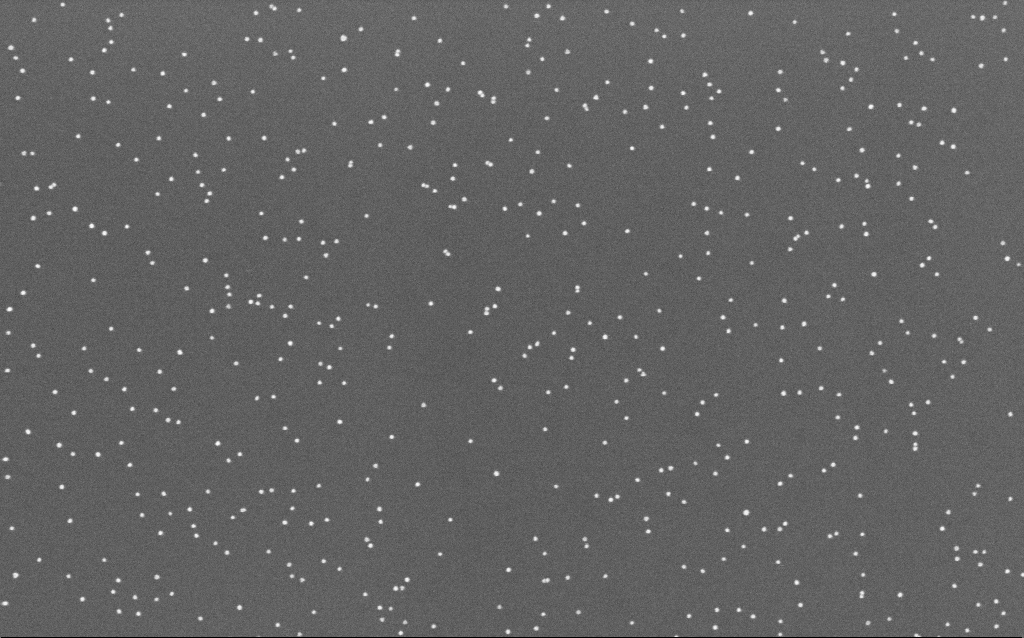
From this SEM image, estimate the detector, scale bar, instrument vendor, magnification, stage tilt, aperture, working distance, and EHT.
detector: InLens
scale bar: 200 nm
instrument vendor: Zeiss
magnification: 100 K X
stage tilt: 0°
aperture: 30 µm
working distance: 6.6 mm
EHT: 10 kV